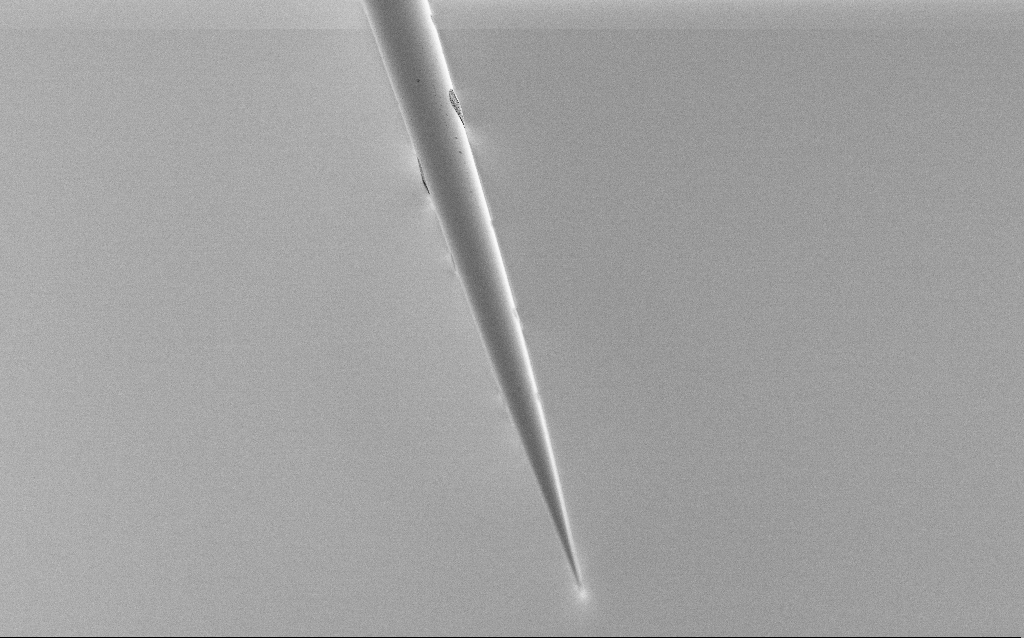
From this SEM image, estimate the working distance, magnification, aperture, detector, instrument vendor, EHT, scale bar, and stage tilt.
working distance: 6 mm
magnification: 1 K X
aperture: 30 µm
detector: SE2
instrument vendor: Zeiss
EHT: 1 kV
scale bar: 20000 nm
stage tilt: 45°